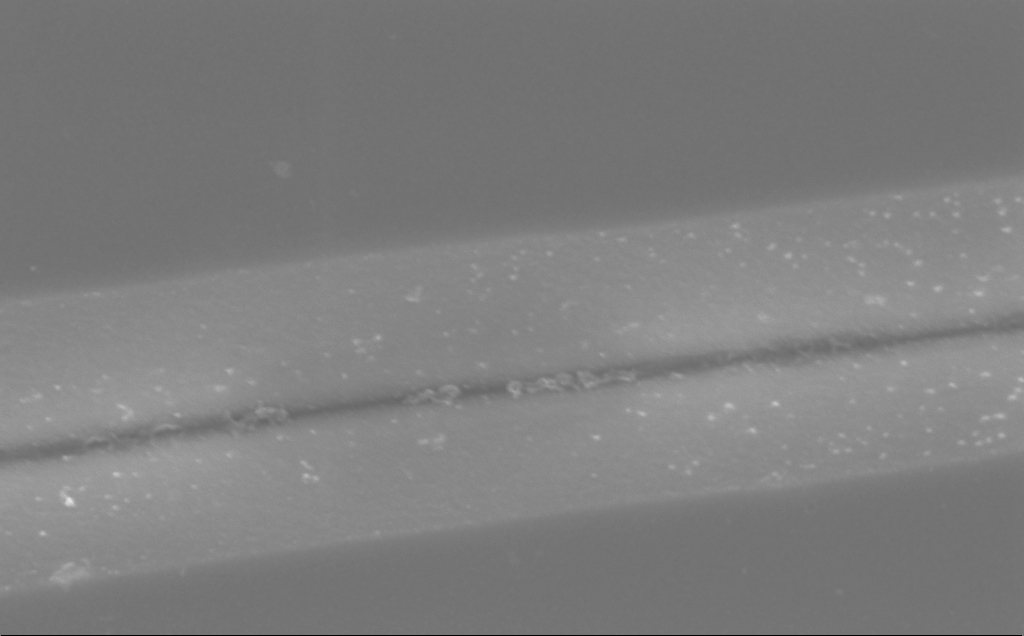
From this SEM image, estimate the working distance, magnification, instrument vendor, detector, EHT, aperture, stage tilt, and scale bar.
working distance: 10 mm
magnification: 59.48 K X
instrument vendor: Zeiss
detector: InLens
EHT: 5 kV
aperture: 30 µm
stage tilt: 0°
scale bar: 1000 nm